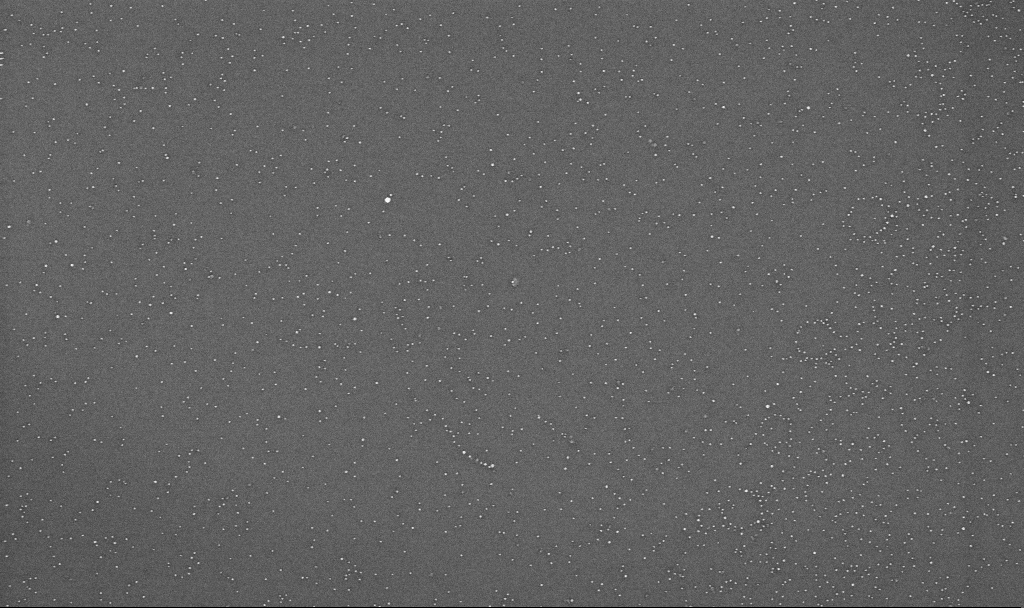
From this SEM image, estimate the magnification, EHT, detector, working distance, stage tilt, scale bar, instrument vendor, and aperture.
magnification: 70 K X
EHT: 10 kV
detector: InLens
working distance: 3.3 mm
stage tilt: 0°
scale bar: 1000 nm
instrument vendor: Zeiss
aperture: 30 µm